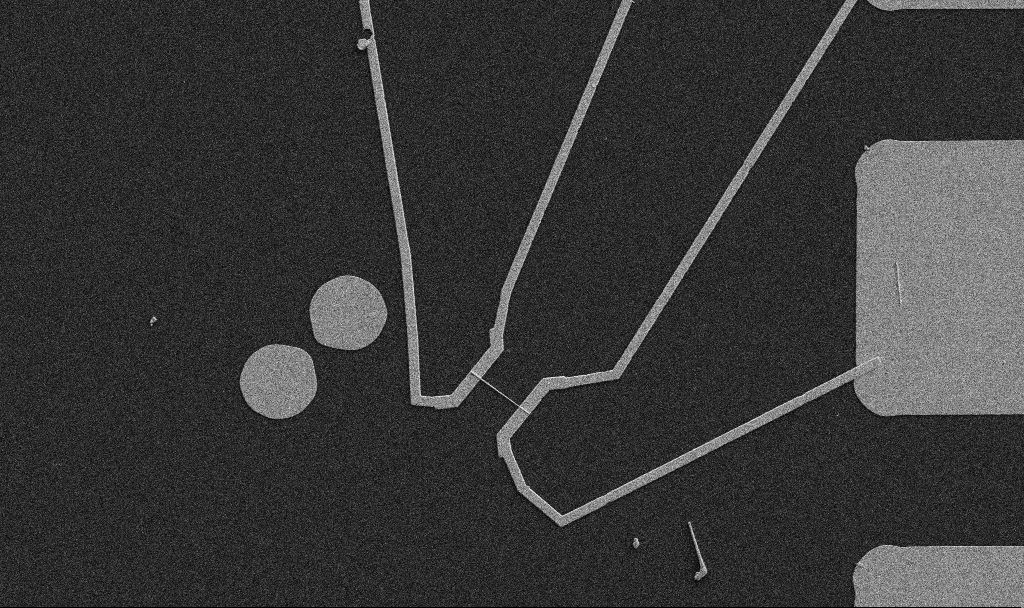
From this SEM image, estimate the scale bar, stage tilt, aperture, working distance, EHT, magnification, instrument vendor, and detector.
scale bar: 10000 nm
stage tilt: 0°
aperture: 30 µm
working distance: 10.7 mm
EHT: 5 kV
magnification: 5 K X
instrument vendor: Zeiss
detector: SE2